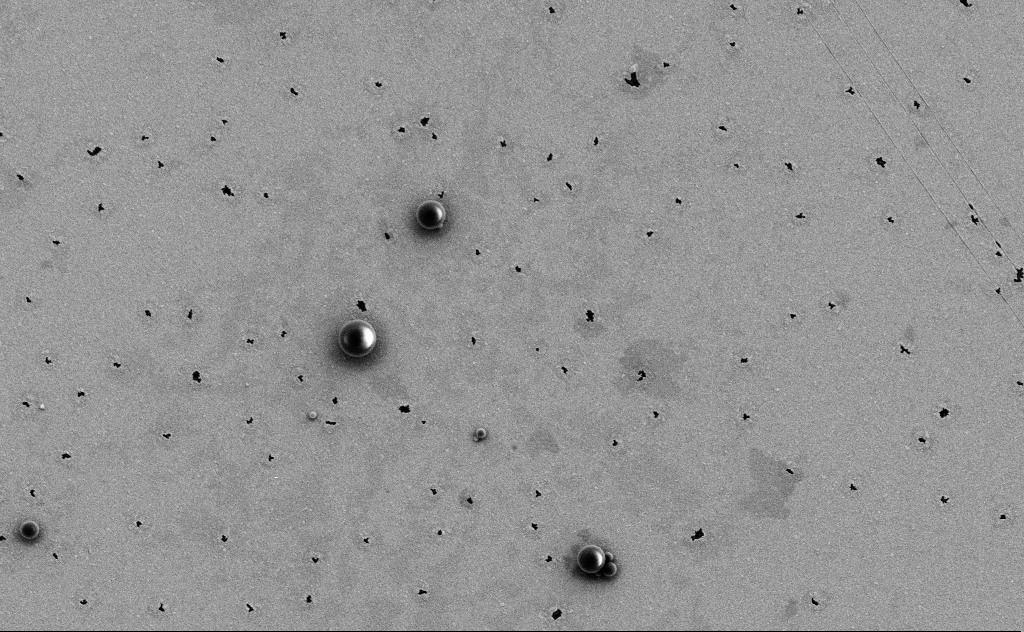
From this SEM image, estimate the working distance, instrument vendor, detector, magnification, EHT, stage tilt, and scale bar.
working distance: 10 mm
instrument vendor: Zeiss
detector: SE2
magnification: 9.65 K X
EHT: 3 kV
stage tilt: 0°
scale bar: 2000 nm